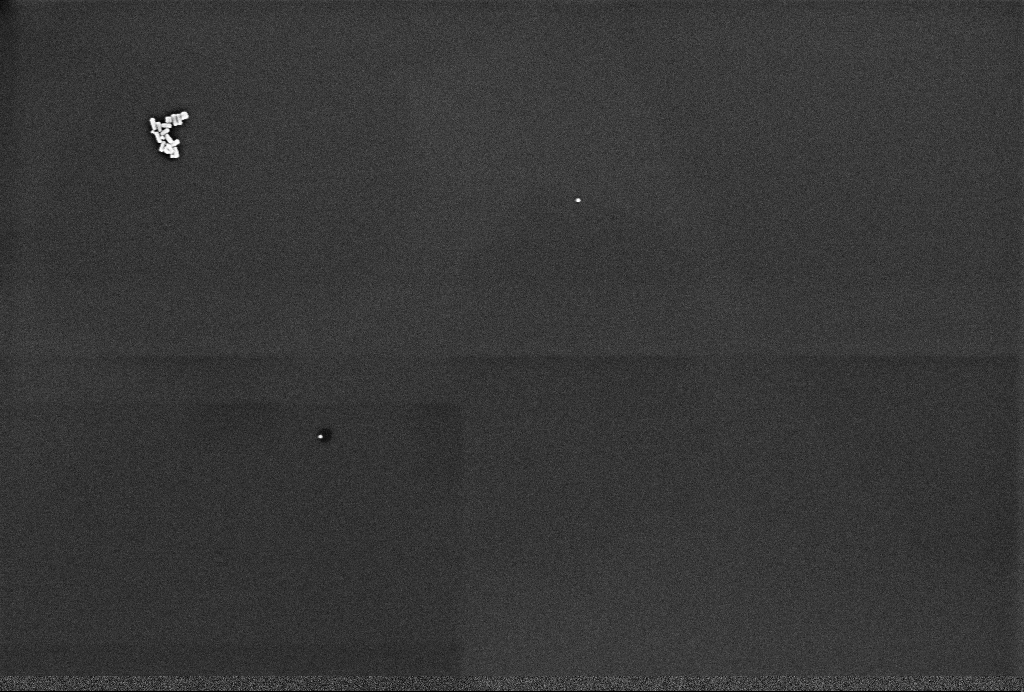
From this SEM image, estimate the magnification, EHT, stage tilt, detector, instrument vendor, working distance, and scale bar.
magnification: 60 K X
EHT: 2 kV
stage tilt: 0°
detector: InLens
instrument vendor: Zeiss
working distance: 3.3 mm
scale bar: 1000 nm